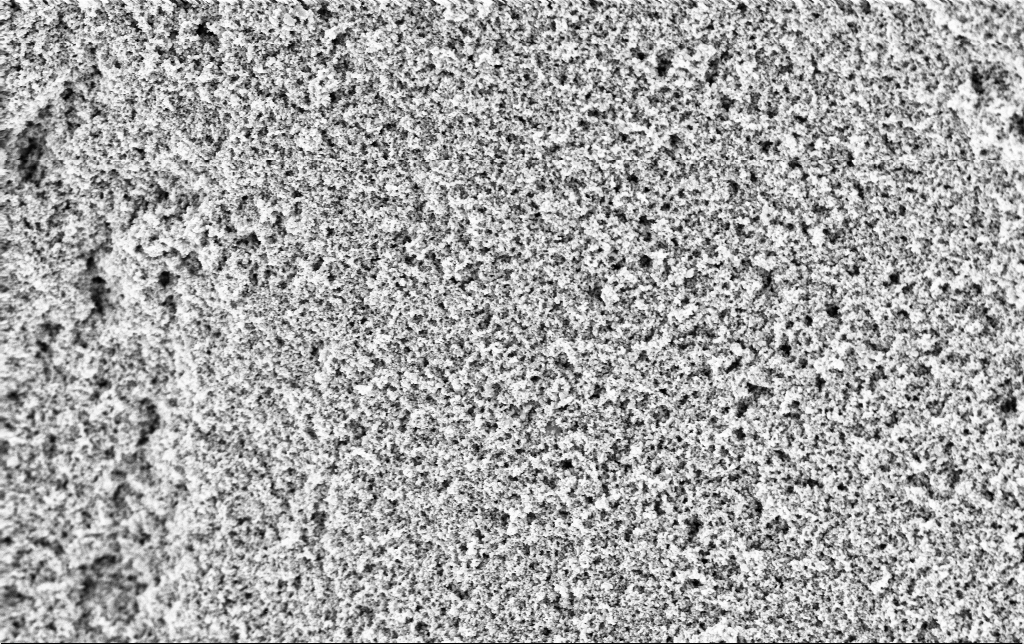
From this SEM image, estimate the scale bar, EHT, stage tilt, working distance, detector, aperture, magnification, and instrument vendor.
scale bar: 1000 nm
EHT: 3 kV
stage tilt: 0°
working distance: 2.4 mm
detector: InLens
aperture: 30 µm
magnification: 30 K X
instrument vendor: Zeiss